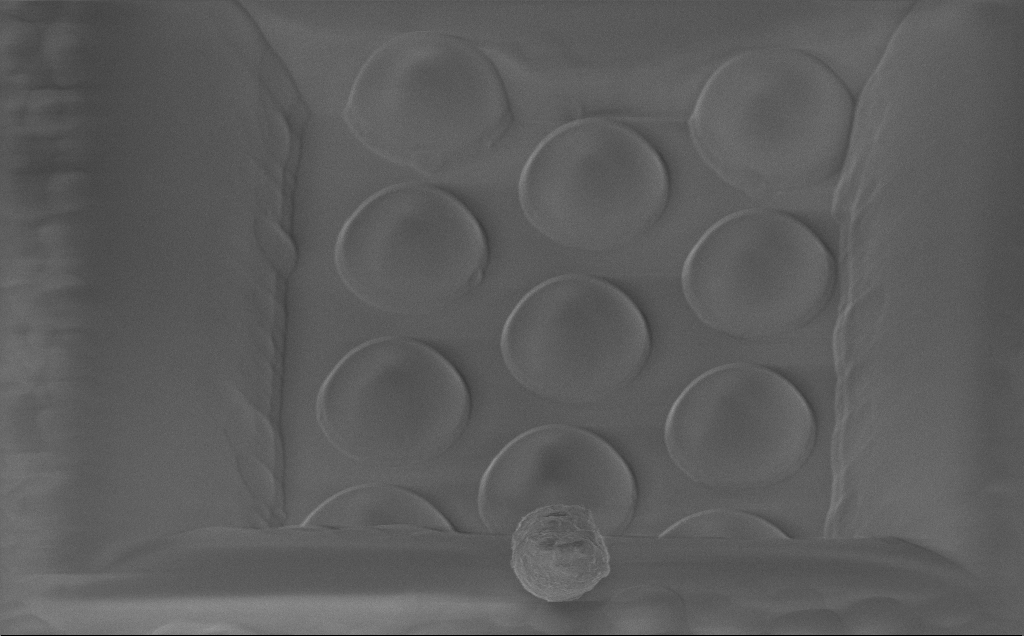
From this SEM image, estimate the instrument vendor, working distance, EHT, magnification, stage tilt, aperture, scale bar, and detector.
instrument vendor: Zeiss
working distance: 6 mm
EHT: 1.3 kV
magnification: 3.53 K X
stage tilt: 38.2°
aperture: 30 µm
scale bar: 20000 nm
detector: InLens